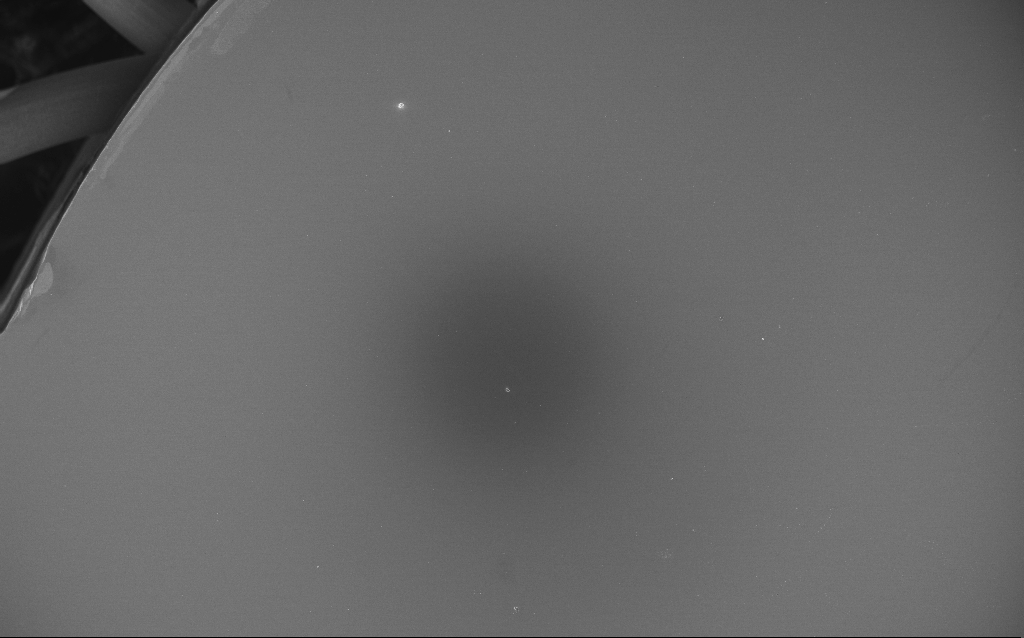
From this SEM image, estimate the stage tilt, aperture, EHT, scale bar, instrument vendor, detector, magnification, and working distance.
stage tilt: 0°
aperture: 30 µm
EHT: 10 kV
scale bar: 100000 nm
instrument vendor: Zeiss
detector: InLens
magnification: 0.173 K X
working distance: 4 mm